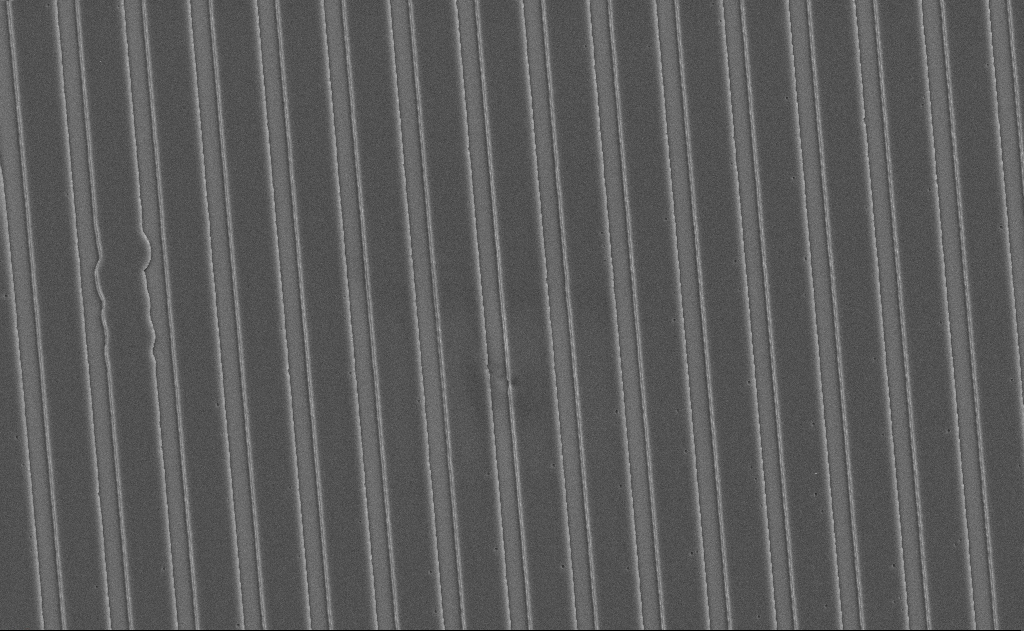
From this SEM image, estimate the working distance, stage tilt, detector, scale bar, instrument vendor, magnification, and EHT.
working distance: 12 mm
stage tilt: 0°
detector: SE2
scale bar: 10000 nm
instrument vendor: Zeiss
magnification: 6.05 K X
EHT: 5 kV